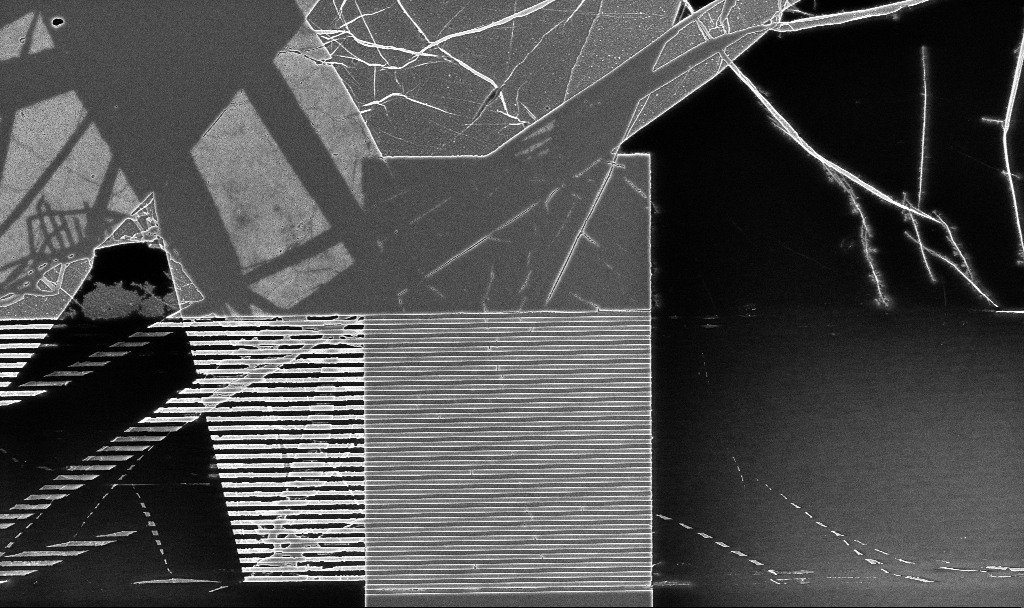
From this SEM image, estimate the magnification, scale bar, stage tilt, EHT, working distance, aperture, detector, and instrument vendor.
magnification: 5.35 K X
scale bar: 10000 nm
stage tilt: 0°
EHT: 5 kV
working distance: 5.2 mm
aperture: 30 µm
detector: InLens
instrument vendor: Zeiss